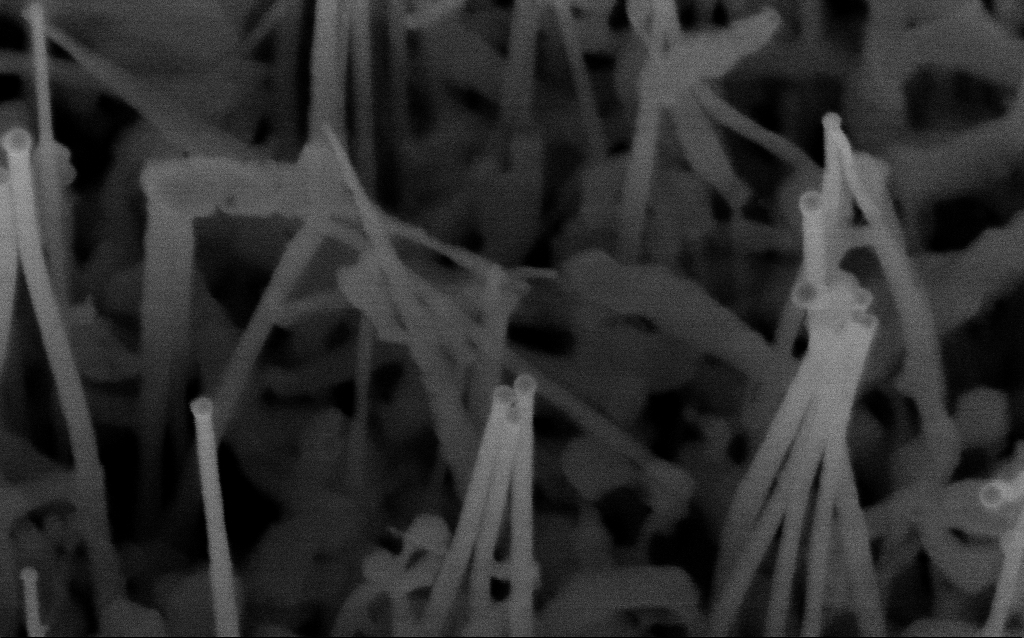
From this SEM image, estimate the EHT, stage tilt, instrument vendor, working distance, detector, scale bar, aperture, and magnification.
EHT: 10 kV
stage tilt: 35°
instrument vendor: Zeiss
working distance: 7.3 mm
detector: InLens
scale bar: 100 nm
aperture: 30 µm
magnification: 264.45 K X